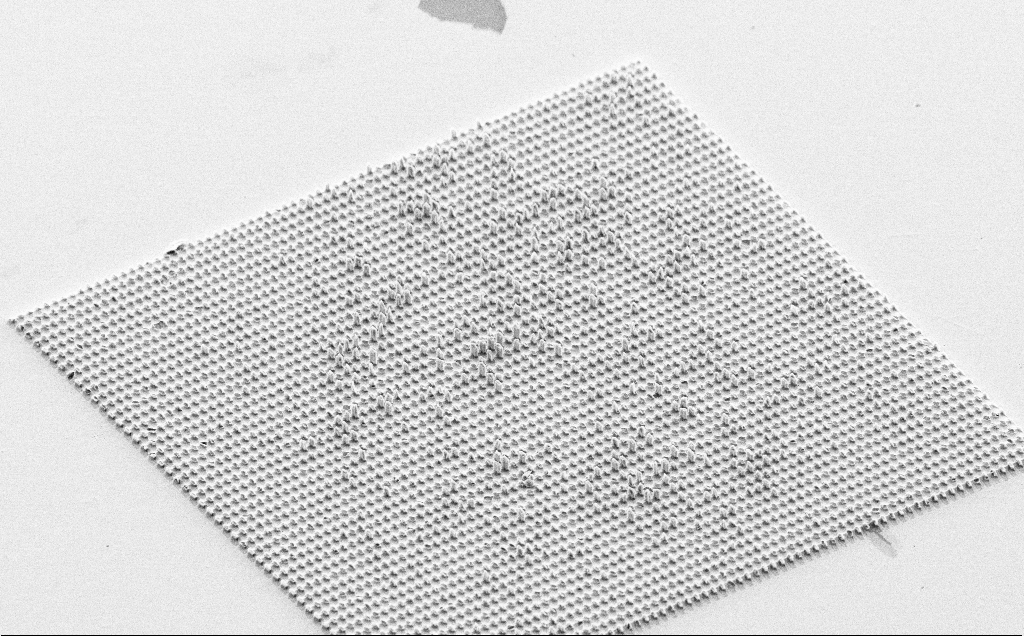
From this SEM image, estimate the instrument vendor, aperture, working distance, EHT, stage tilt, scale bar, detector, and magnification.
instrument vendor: Zeiss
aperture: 30 µm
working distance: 9 mm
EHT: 10 kV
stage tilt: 51.2°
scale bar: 20000 nm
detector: SE2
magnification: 0.942 K X